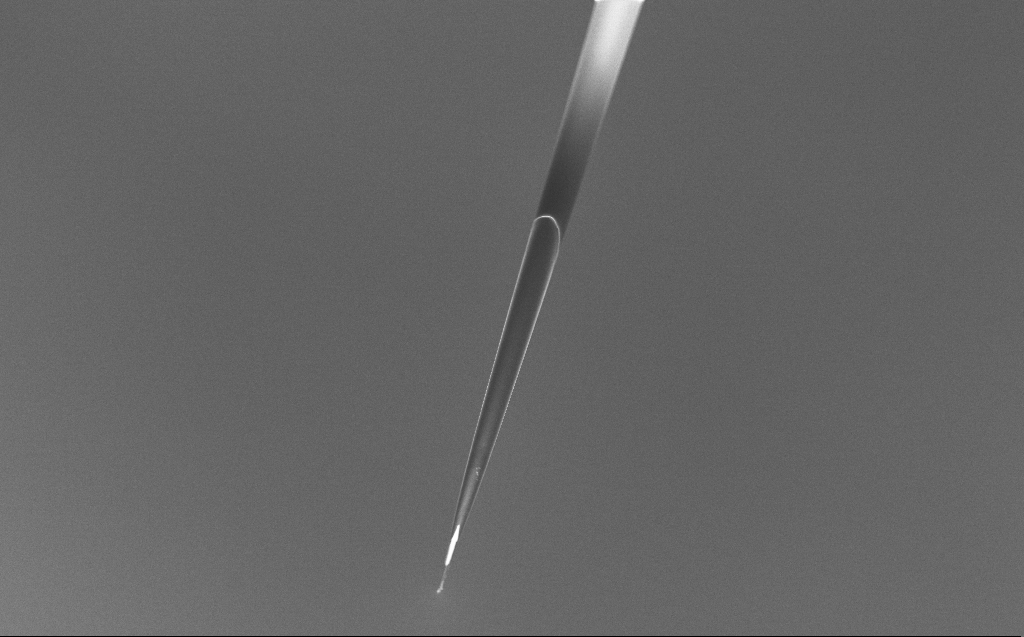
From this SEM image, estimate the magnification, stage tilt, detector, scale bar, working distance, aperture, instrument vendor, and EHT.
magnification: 0.5 K X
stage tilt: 45°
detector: InLens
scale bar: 100000 nm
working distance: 6 mm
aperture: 30 µm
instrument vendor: Zeiss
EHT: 5 kV